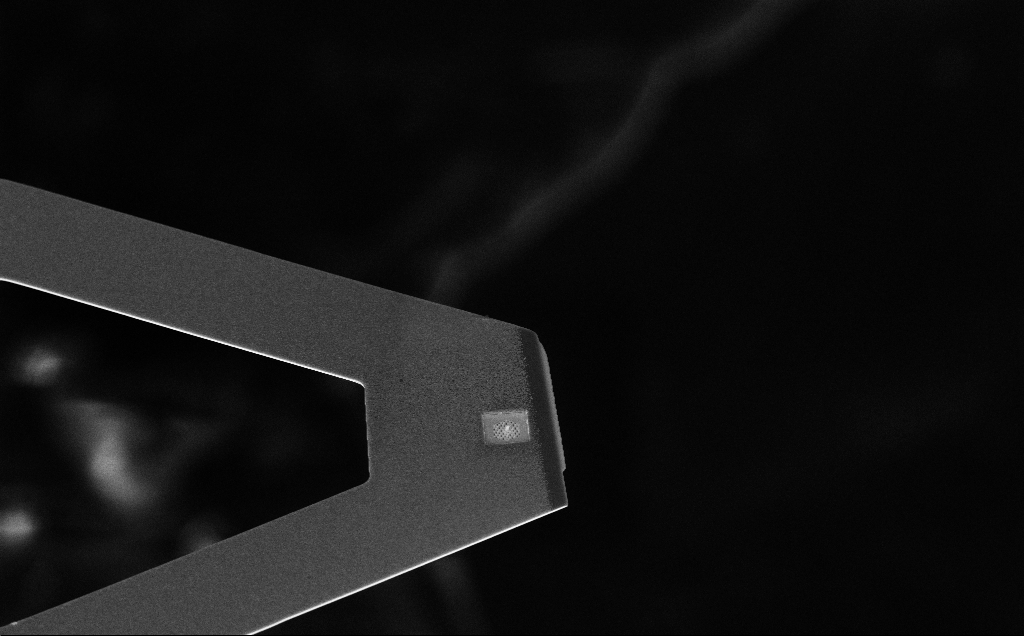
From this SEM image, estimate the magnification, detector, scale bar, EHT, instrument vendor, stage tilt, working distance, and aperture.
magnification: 2.6 K X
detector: InLens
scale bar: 10000 nm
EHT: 10 kV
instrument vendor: Zeiss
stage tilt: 45°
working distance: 11 mm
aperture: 30 µm